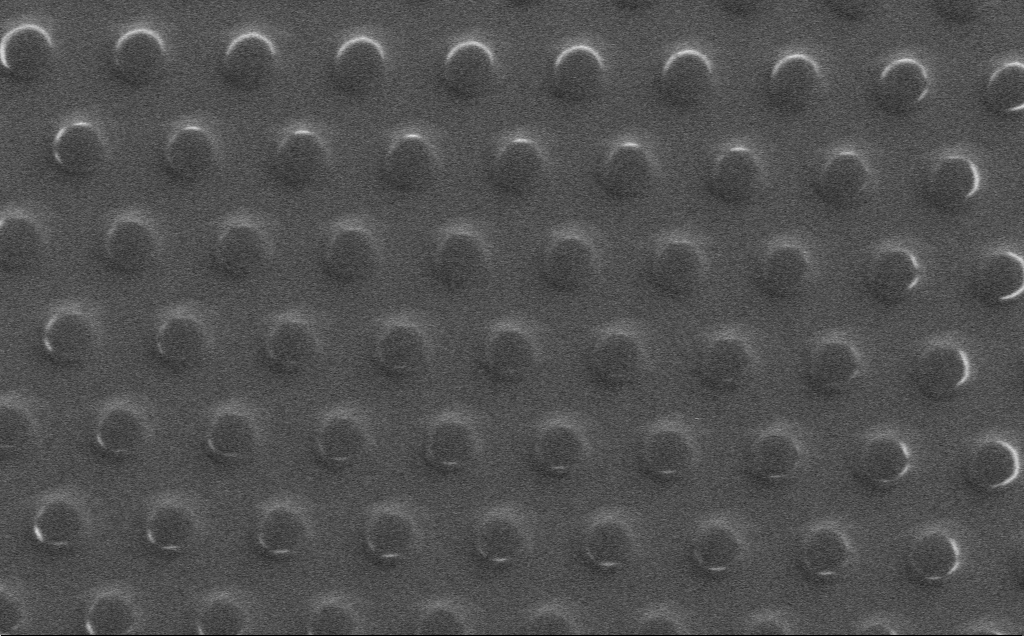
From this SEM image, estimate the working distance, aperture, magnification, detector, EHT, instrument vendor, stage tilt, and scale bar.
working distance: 4 mm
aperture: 30 µm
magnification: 2.04 K X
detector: SE2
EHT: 3 kV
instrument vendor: Zeiss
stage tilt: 0°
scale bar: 10000 nm